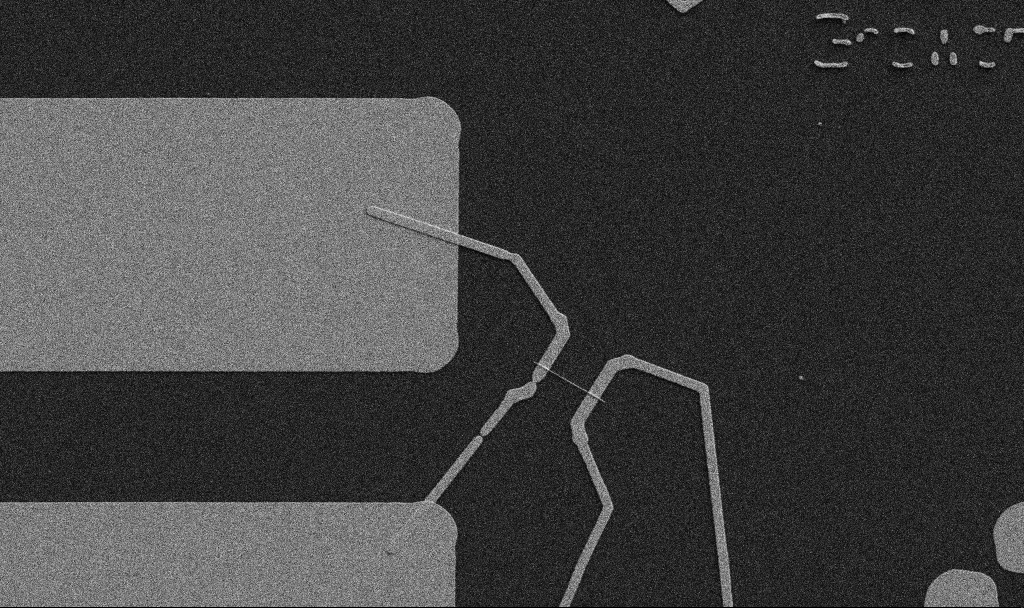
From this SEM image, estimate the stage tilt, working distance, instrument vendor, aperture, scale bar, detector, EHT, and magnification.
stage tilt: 0°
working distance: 10.7 mm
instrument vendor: Zeiss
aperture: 30 µm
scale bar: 10000 nm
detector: SE2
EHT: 5 kV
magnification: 5 K X